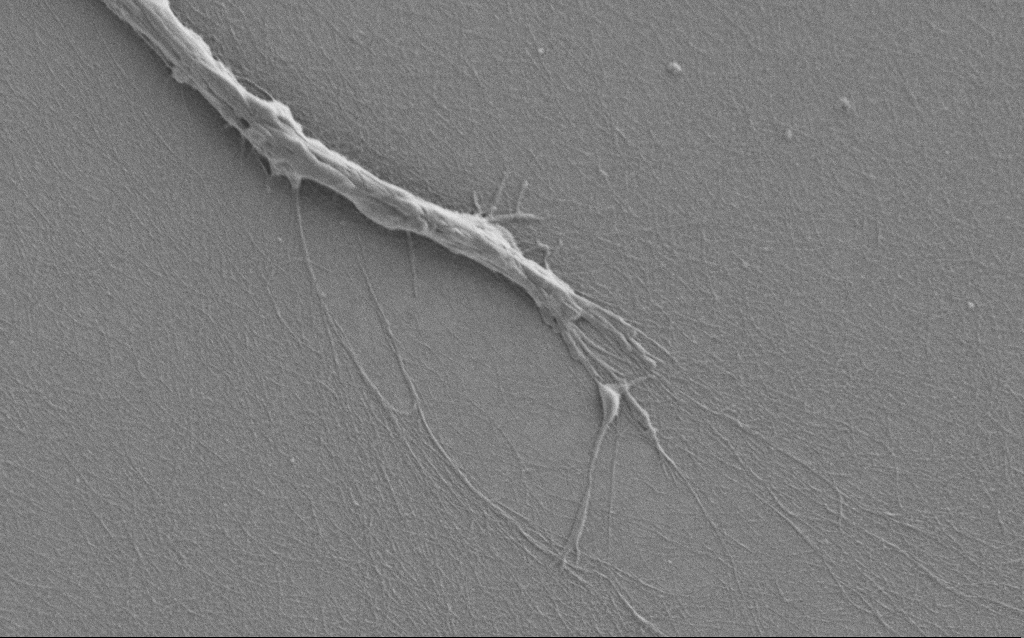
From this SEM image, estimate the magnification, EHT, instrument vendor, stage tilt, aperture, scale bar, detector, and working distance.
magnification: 7.5 K X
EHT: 1 kV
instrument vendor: Zeiss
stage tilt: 0°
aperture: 30 µm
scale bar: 2000 nm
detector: SE2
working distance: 6 mm